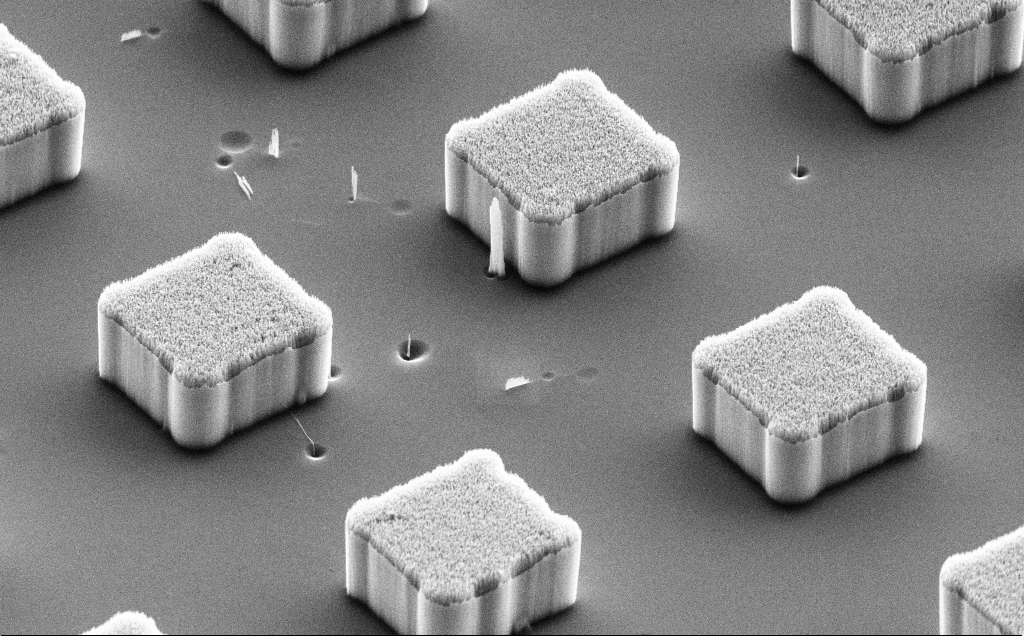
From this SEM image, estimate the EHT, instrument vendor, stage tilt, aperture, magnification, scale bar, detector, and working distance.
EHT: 10 kV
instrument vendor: Zeiss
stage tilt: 50.6°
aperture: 30 µm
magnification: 7.81 K X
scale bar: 2000 nm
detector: SE2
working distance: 13 mm